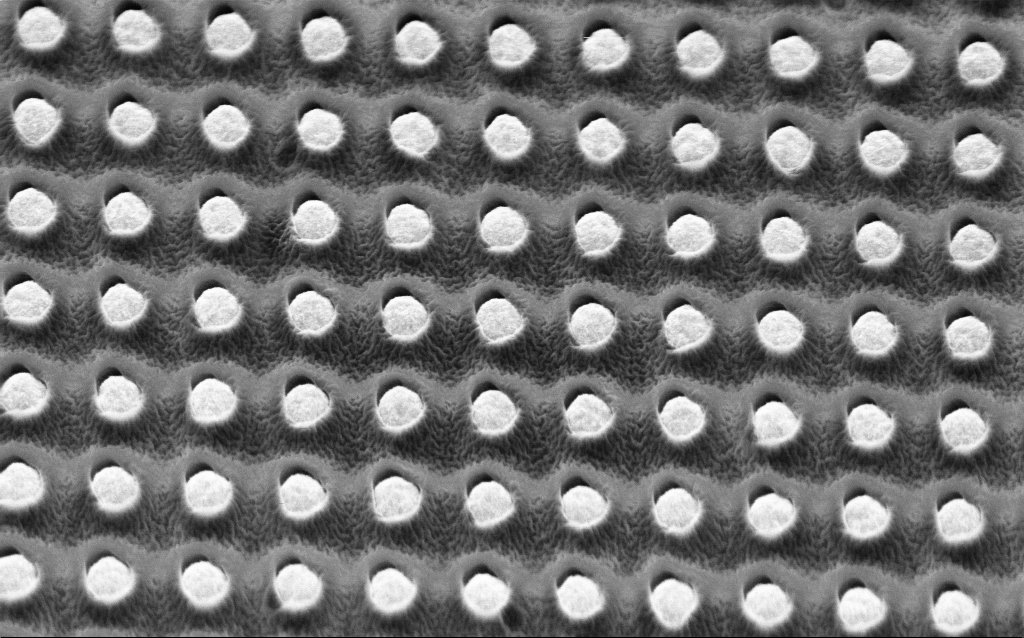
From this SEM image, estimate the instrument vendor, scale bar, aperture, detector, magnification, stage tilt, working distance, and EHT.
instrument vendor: Zeiss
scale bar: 1000 nm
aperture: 30 µm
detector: InLens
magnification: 69.48 K X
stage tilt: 45°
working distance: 3.5 mm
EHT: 2 kV